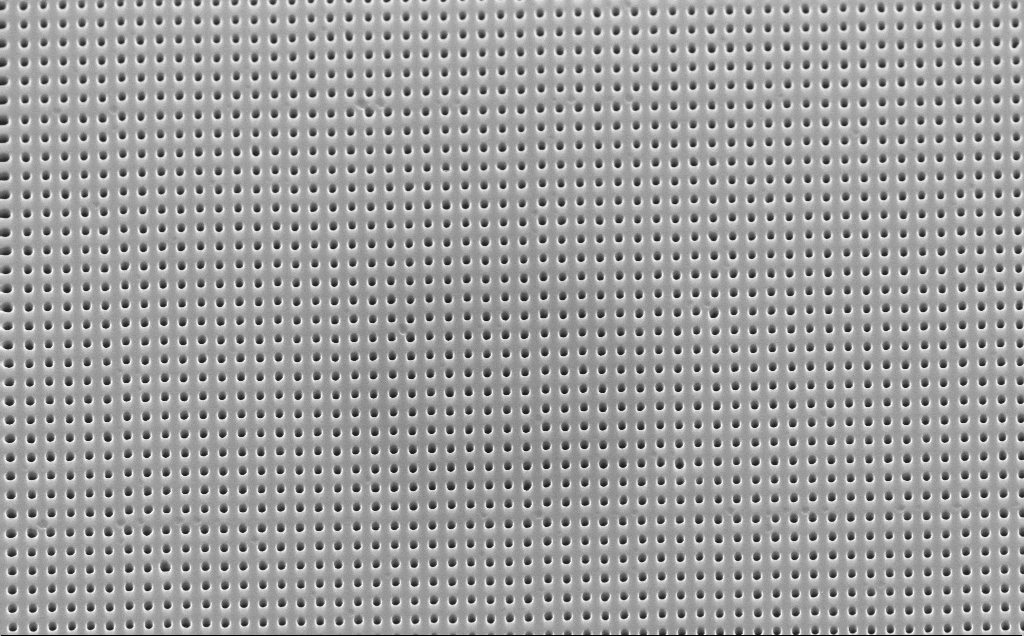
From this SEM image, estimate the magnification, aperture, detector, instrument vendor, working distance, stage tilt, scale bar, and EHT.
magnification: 35 K X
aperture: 30 µm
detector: InLens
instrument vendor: Zeiss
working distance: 4 mm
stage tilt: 30°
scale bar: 1000 nm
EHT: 10 kV